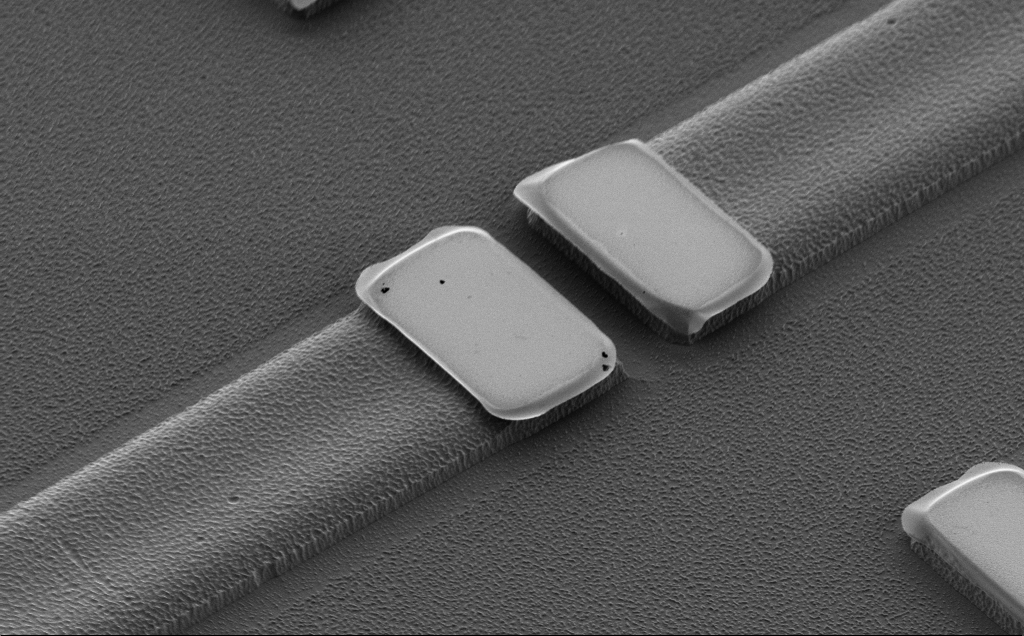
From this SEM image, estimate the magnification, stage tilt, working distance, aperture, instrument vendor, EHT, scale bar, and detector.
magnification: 5.47 K X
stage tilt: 43°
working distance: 10 mm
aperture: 30 µm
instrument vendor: Zeiss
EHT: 2 kV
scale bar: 10000 nm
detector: SE2